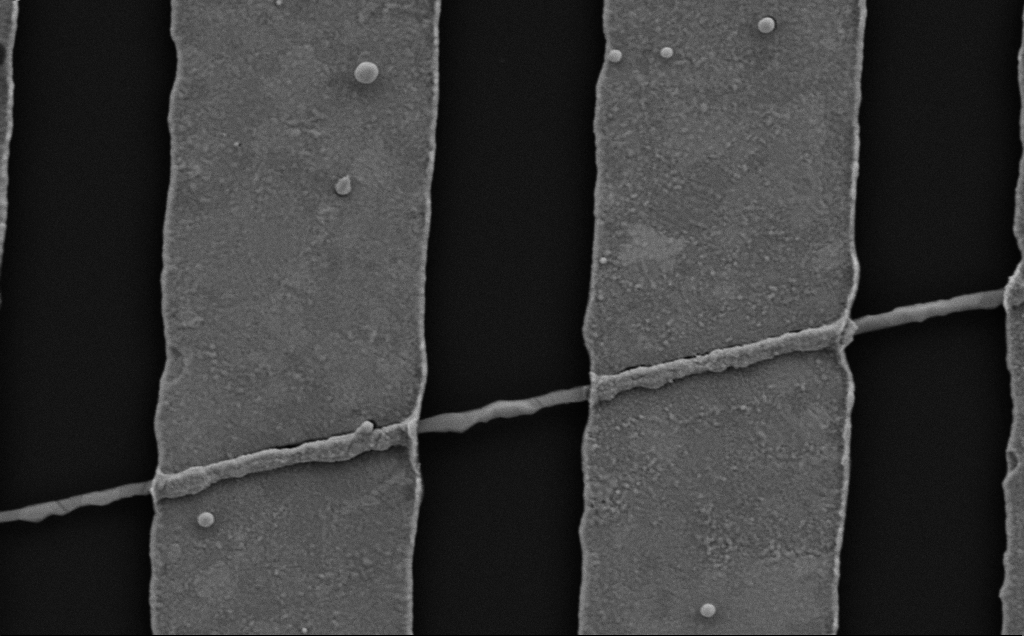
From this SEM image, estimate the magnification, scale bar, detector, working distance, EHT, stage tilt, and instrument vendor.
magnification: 39.46 K X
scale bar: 1000 nm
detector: SE2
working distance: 6 mm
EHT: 5 kV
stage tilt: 0°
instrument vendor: Zeiss